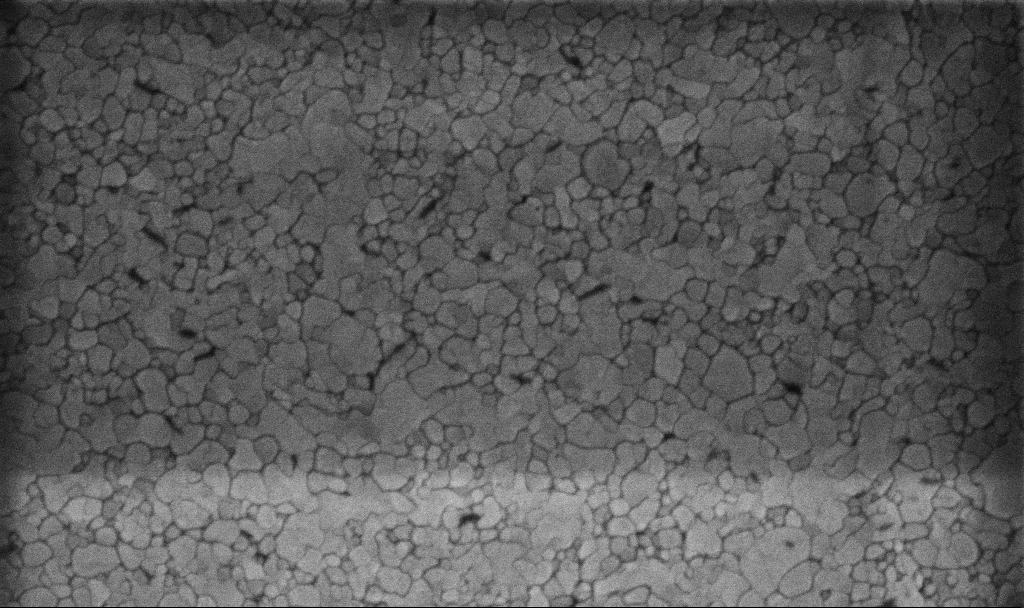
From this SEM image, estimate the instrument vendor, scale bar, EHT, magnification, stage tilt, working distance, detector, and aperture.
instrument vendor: Zeiss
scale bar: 200 nm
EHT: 5 kV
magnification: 100.15 K X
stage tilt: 0°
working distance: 3.1 mm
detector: InLens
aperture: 30 µm